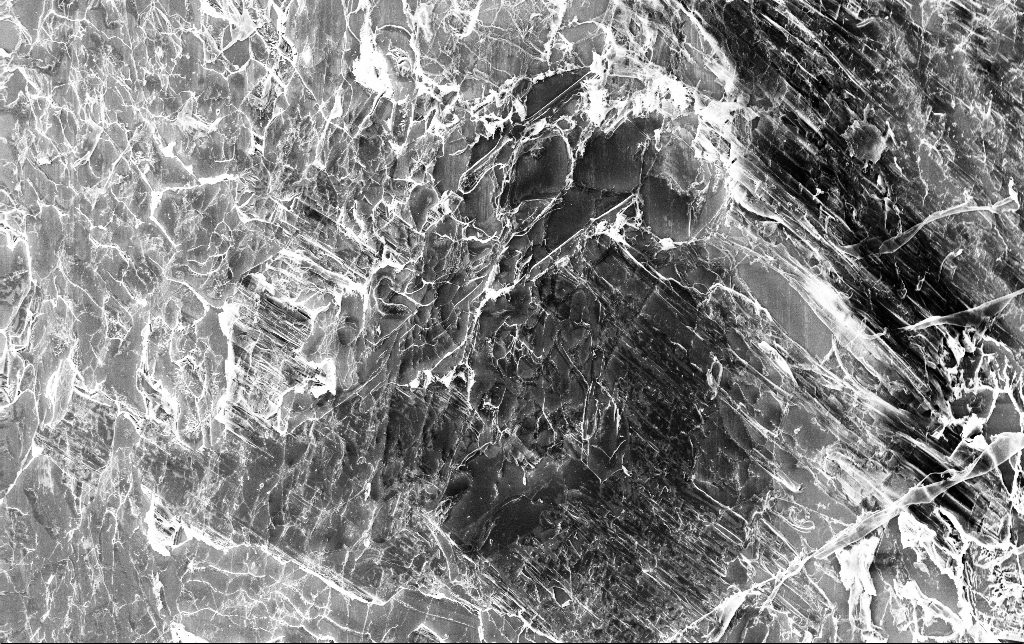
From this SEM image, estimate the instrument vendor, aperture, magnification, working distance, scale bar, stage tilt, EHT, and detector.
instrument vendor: Zeiss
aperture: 30 µm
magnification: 0.397 K X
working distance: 3.4 mm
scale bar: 100000 nm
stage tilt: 0°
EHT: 10 kV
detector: InLens